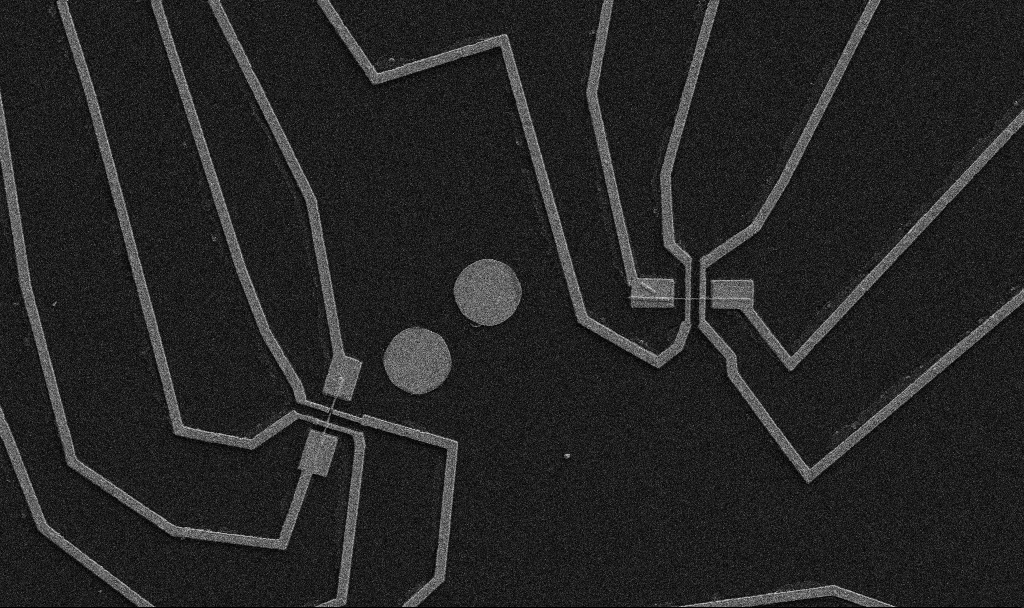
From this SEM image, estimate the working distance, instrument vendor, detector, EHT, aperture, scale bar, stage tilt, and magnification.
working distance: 10.7 mm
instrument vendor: Zeiss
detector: SE2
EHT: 5 kV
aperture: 30 µm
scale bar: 10000 nm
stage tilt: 0°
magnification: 5 K X